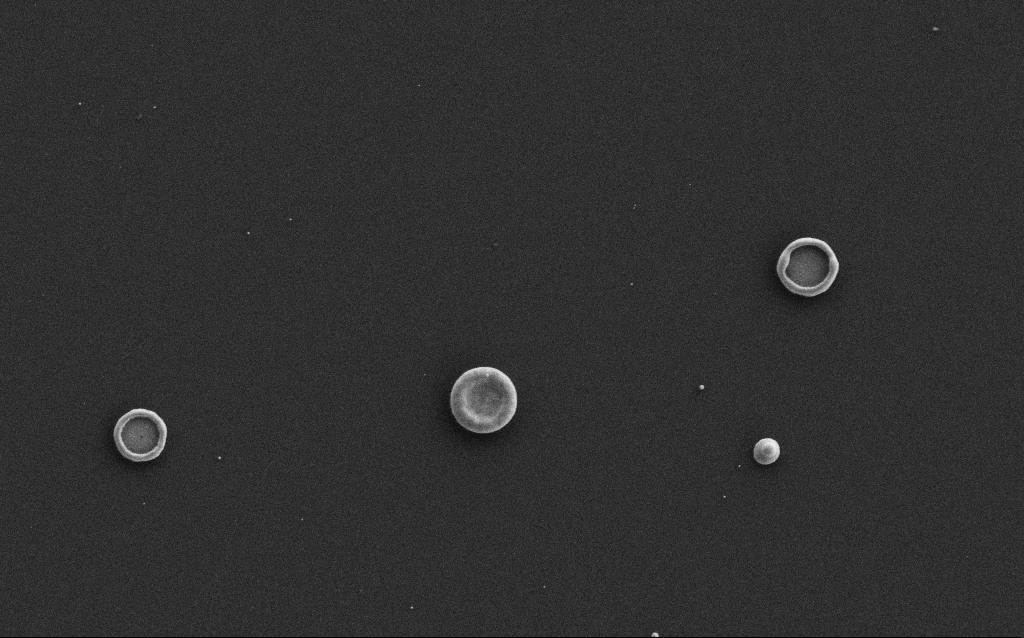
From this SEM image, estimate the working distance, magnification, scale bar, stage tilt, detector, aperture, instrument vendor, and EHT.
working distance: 3 mm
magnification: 11 K X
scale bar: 2000 nm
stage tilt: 0°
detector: SE2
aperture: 30 µm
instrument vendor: Zeiss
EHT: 3 kV